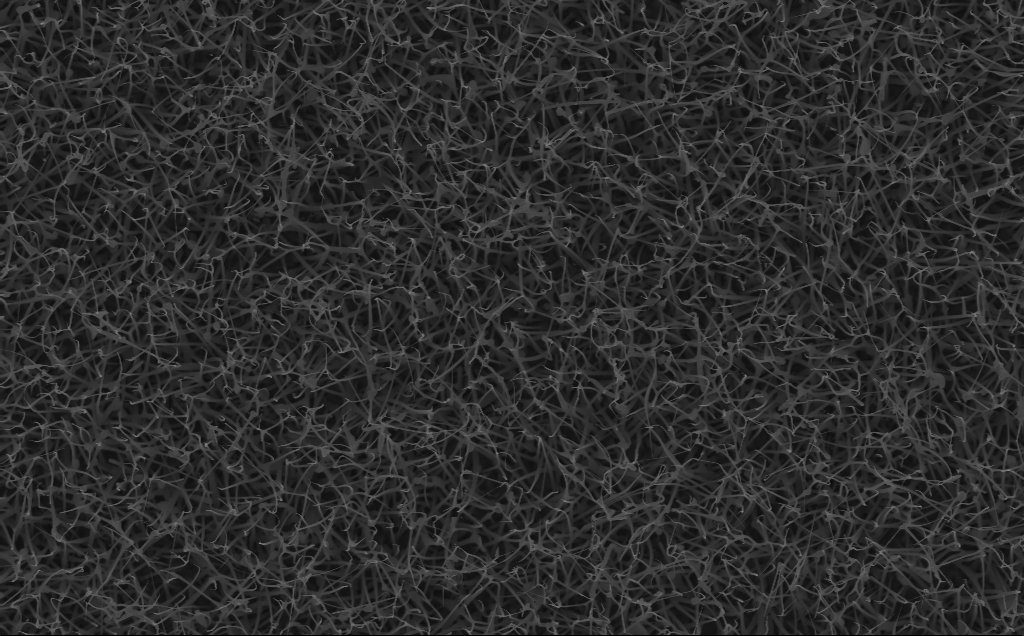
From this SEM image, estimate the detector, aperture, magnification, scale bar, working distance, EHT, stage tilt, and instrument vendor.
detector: InLens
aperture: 30 µm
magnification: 10 K X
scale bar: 2000 nm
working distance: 6 mm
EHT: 10 kV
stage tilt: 0°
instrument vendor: Zeiss